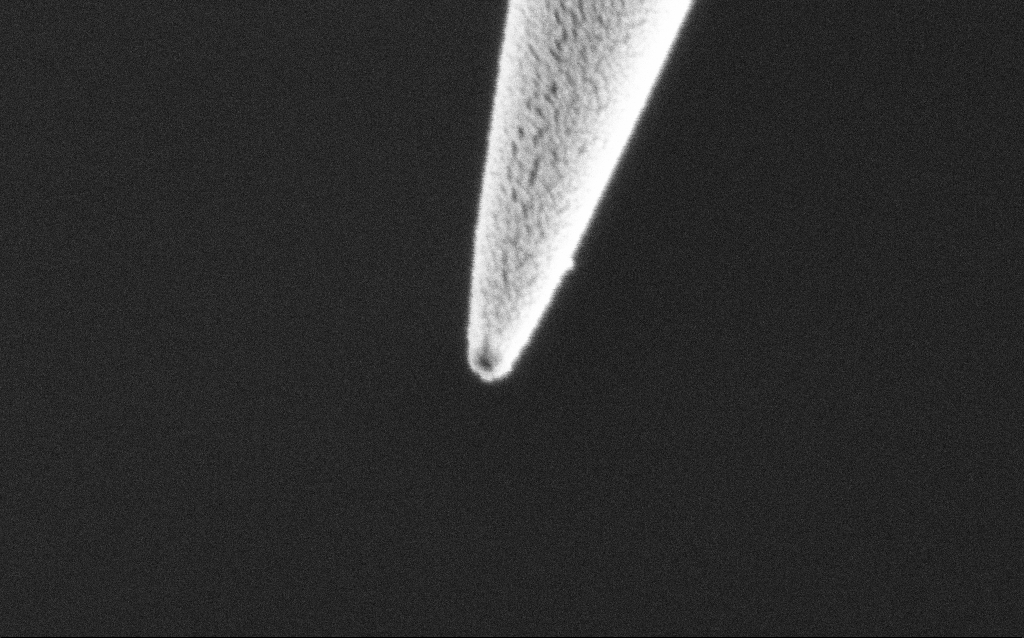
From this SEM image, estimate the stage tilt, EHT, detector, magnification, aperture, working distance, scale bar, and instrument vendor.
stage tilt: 45°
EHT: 2 kV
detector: SE2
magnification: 150 K X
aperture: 30 µm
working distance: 7.6 mm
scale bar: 200 nm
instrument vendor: Zeiss